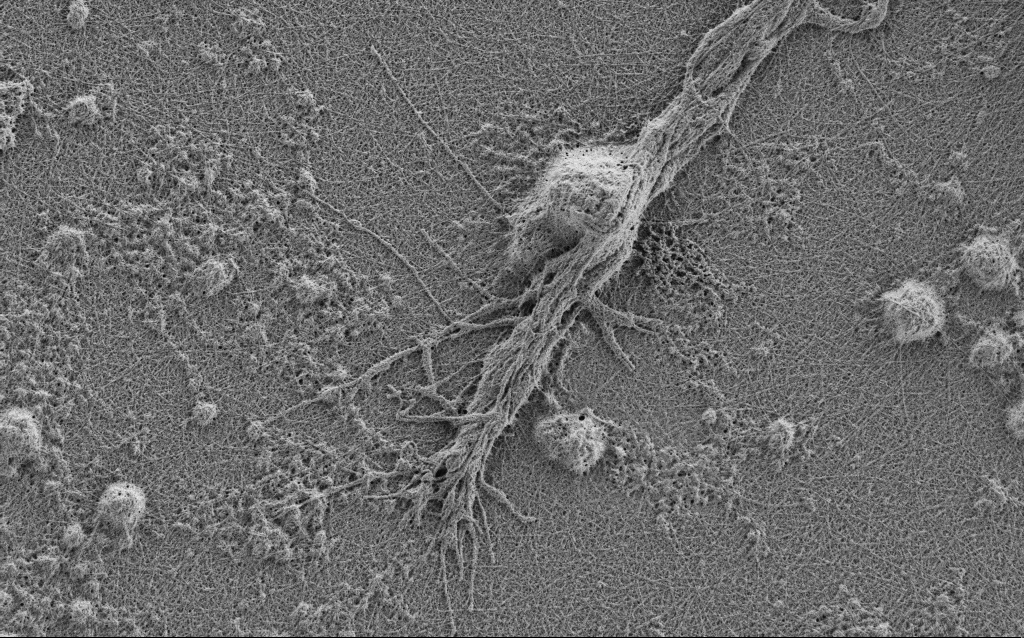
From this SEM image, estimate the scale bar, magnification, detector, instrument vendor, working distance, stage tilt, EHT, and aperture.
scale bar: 2000 nm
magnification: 10 K X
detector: SE2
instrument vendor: Zeiss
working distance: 4 mm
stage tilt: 0°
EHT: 0.9 kV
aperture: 30 µm